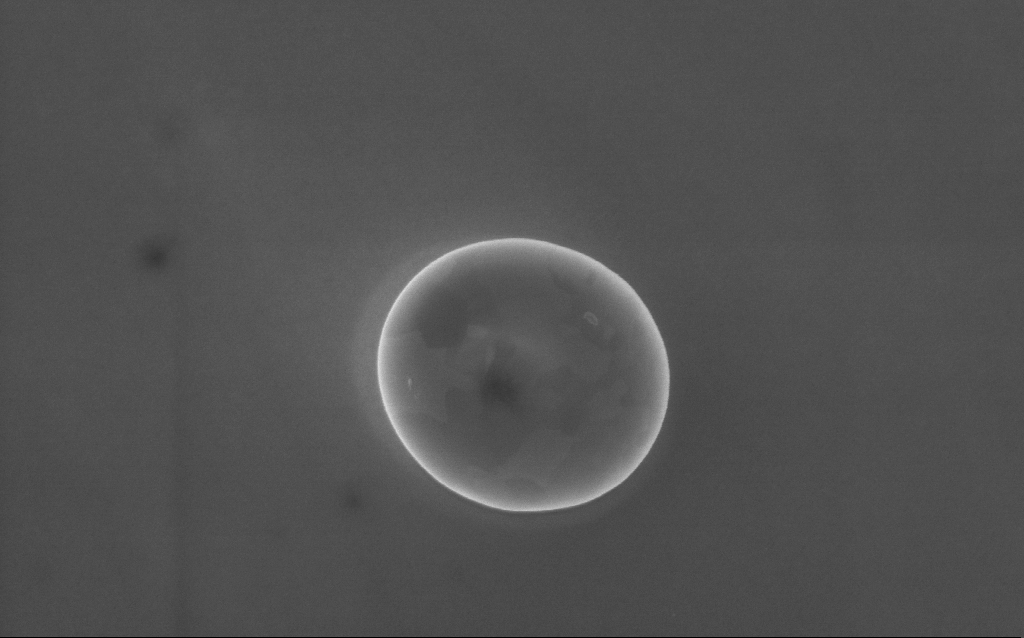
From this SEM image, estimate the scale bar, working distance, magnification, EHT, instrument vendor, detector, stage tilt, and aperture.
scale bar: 1000 nm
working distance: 4 mm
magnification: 62 K X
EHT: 5 kV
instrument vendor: Zeiss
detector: InLens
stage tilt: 0°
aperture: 30 µm